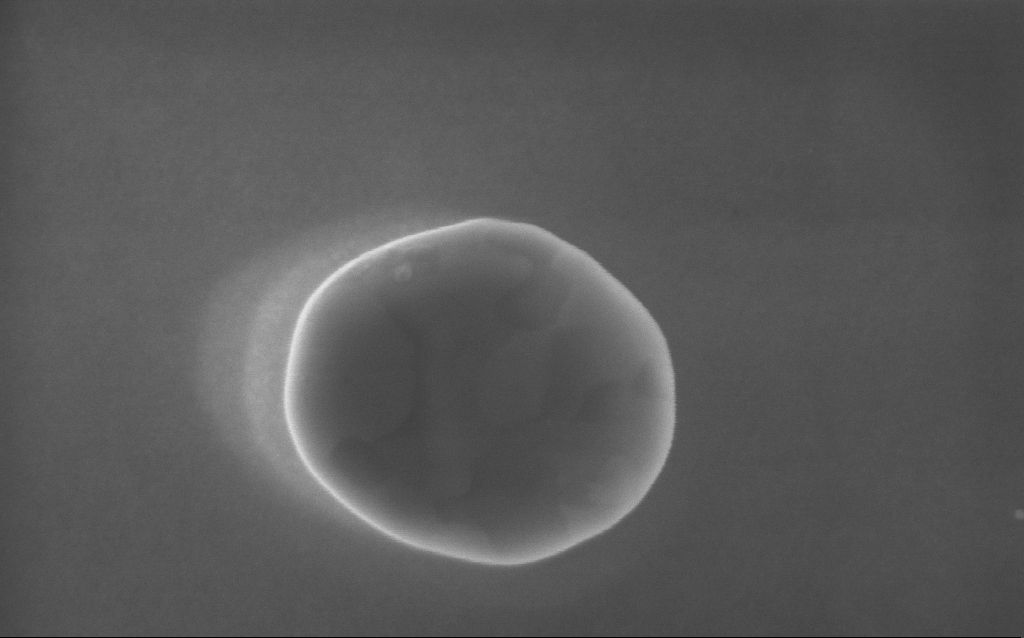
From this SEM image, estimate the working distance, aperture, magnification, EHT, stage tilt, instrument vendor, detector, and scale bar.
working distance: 4 mm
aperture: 30 µm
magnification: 151 K X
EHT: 5 kV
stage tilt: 0°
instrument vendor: Zeiss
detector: InLens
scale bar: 200 nm